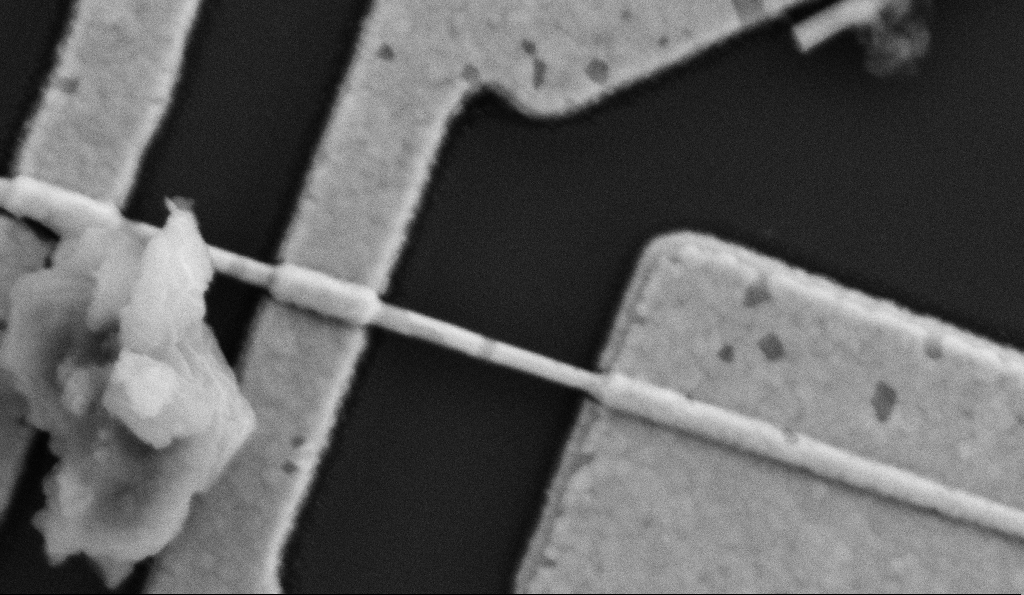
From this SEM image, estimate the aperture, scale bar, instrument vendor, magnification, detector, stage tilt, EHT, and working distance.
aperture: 30 µm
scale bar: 200 nm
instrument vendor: Zeiss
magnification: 100 K X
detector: SE2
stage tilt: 0°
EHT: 5 kV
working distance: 9.5 mm